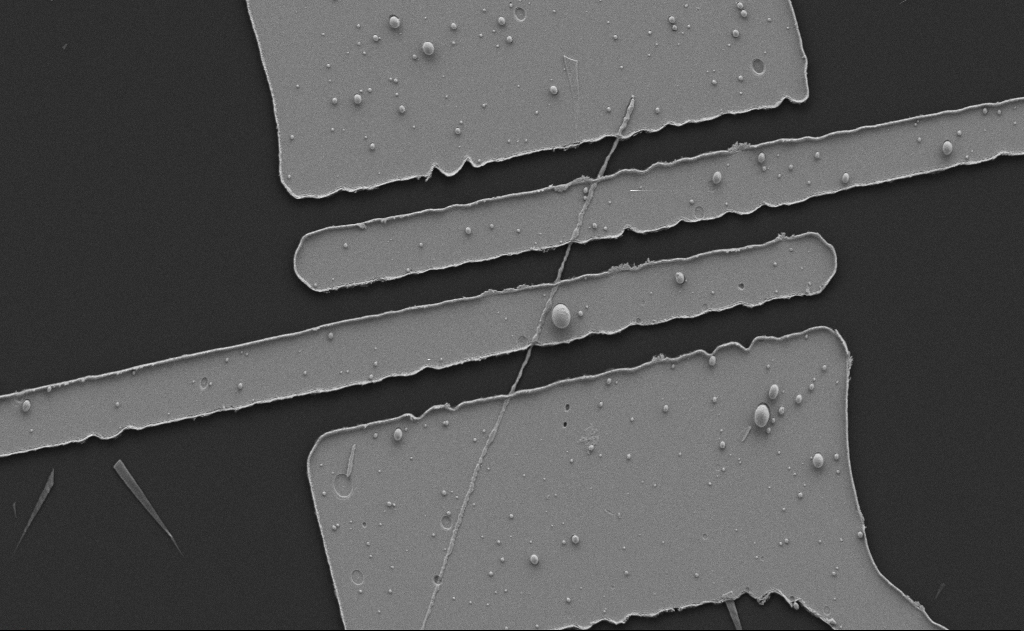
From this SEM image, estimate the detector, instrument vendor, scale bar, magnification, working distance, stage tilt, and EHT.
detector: SE2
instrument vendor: Zeiss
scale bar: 10000 nm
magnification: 6.72 K X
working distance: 10 mm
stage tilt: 0°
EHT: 5 kV